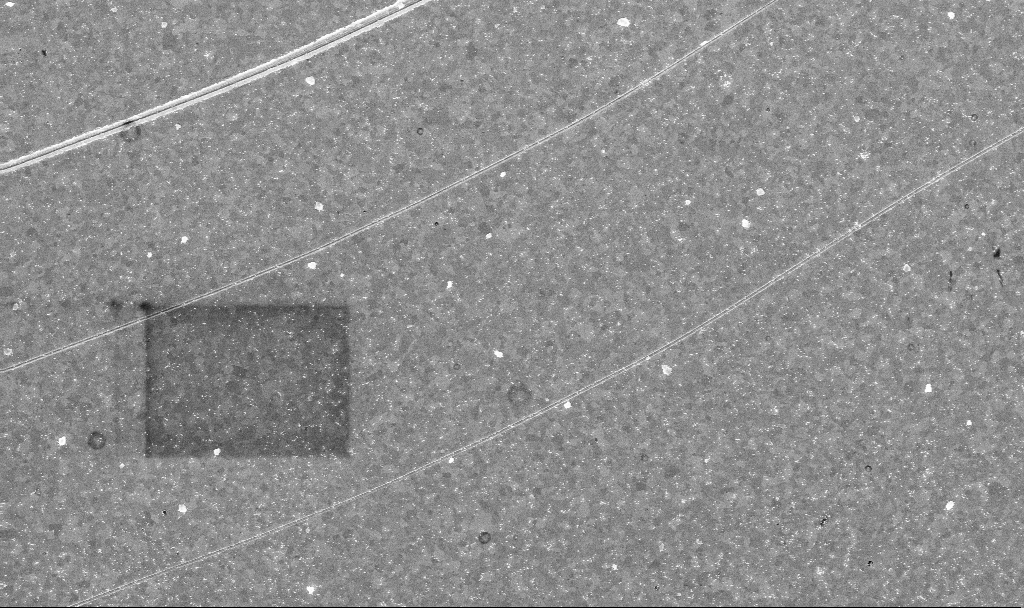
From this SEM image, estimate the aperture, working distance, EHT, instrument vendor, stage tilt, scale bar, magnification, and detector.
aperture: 30 µm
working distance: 3.4 mm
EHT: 10 kV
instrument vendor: Zeiss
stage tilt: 0°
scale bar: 2000 nm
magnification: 10.04 K X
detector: InLens